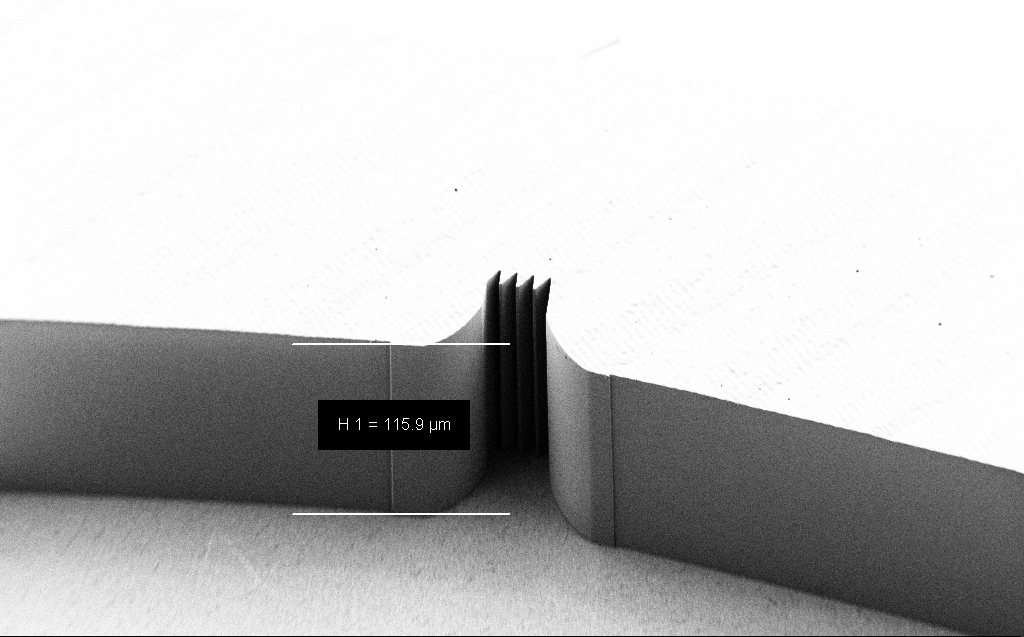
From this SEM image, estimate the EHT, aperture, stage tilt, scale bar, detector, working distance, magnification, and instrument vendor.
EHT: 1 kV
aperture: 30 µm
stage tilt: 45°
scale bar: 100000 nm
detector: SE2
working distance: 7 mm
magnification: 0.539 K X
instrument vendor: Zeiss